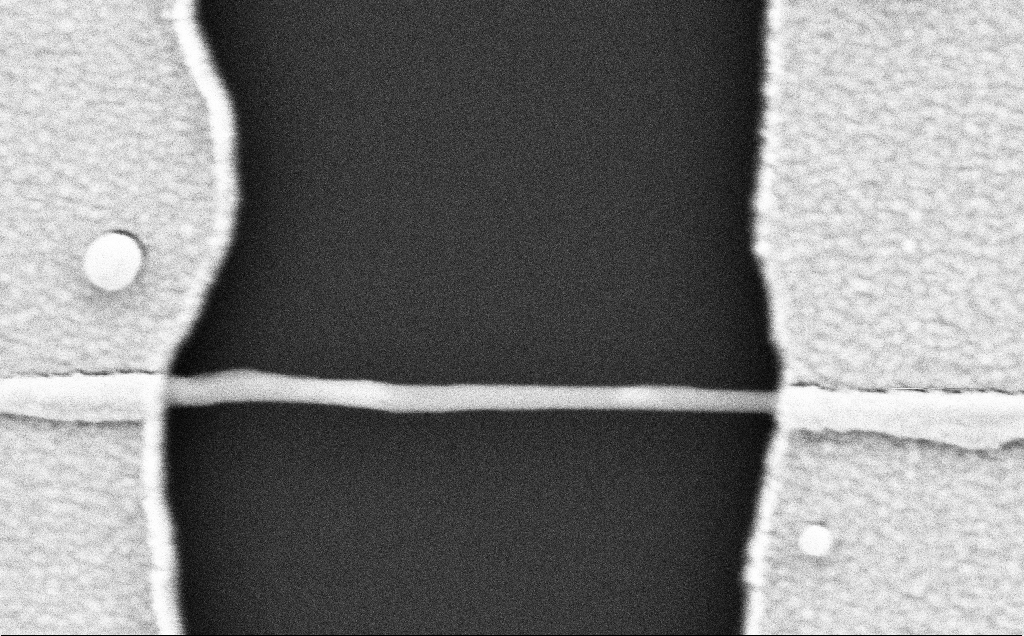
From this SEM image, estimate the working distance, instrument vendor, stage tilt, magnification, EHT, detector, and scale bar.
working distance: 10 mm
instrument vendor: Zeiss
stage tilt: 0°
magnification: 126.61 K X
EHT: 5 kV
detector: SE2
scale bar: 200 nm